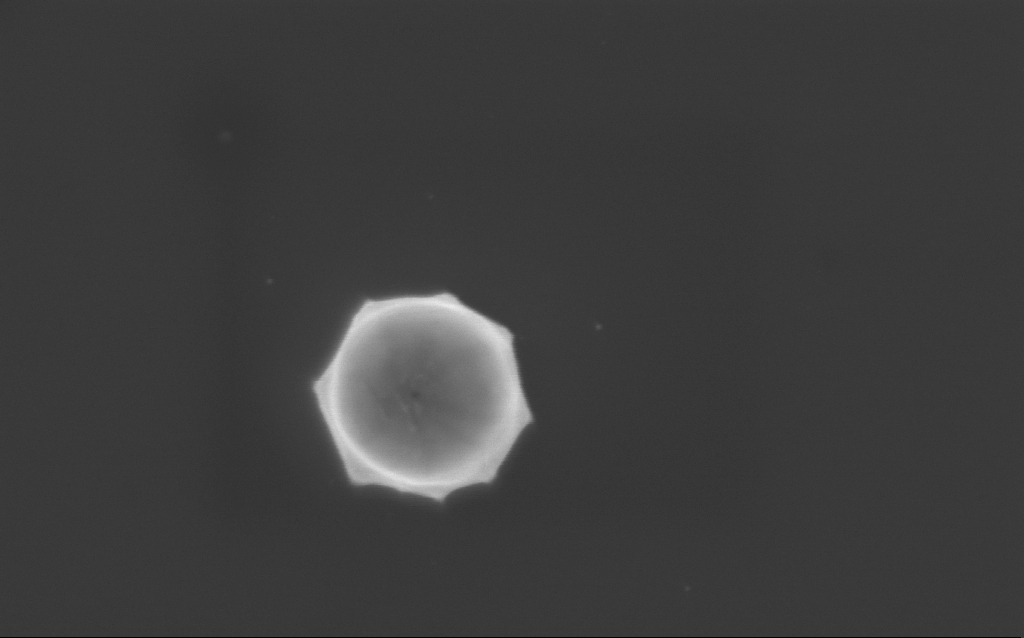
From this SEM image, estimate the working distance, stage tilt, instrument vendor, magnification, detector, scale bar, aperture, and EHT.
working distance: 3 mm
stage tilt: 0°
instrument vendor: Zeiss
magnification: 134.29 K X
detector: InLens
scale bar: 200 nm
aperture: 30 µm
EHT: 3 kV